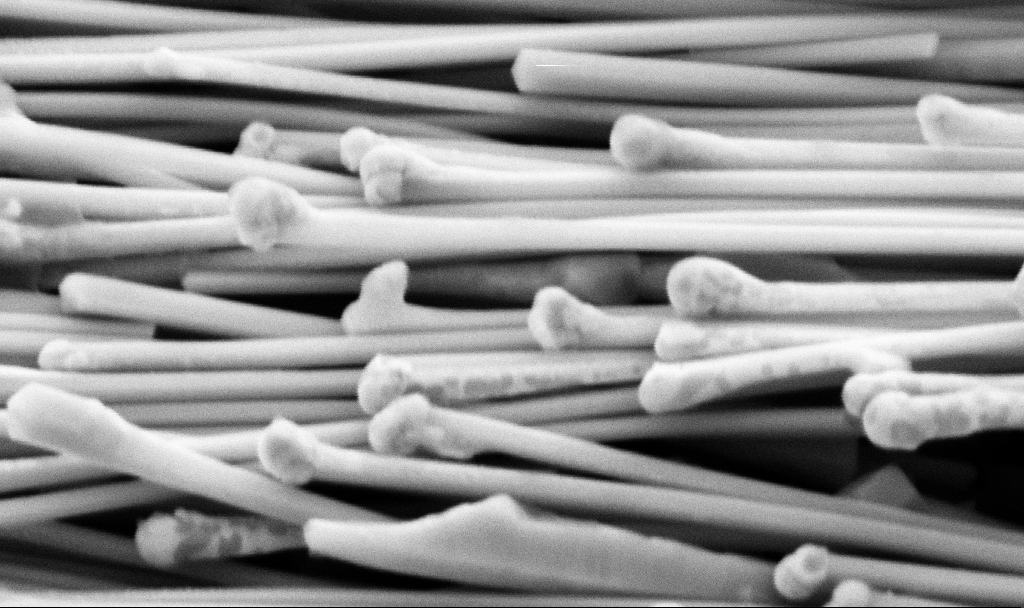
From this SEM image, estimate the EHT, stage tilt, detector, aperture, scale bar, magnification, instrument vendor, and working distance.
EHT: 5 kV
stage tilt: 45°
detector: SE2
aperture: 30 µm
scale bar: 200 nm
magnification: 94.55 K X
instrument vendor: Zeiss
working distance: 11.2 mm